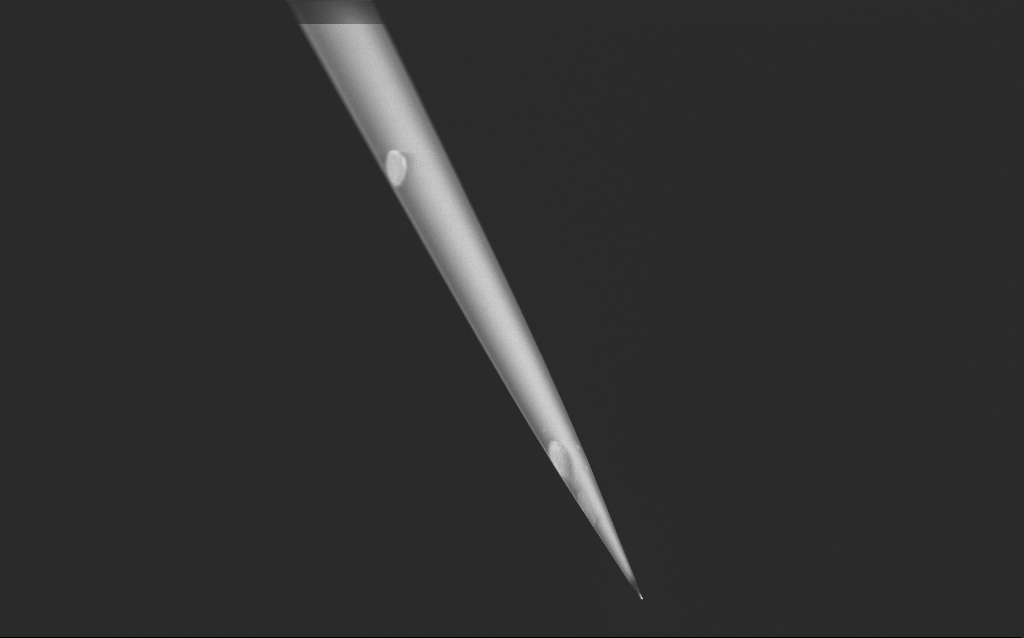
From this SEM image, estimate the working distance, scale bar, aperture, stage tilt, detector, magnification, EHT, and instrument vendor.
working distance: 6 mm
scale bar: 20000 nm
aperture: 30 µm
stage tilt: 45°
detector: InLens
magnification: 1 K X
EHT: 1 kV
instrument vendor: Zeiss